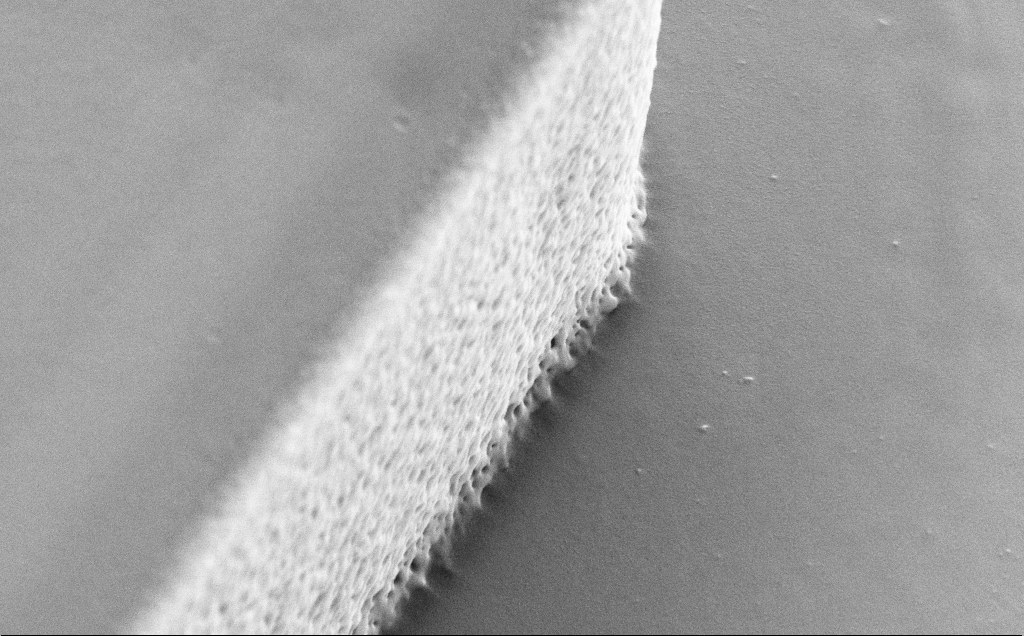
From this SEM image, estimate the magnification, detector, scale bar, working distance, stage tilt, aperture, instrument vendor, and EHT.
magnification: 33.05 K X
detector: SE2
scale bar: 2000 nm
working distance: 9 mm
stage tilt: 30°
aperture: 30 µm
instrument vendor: Zeiss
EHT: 5 kV